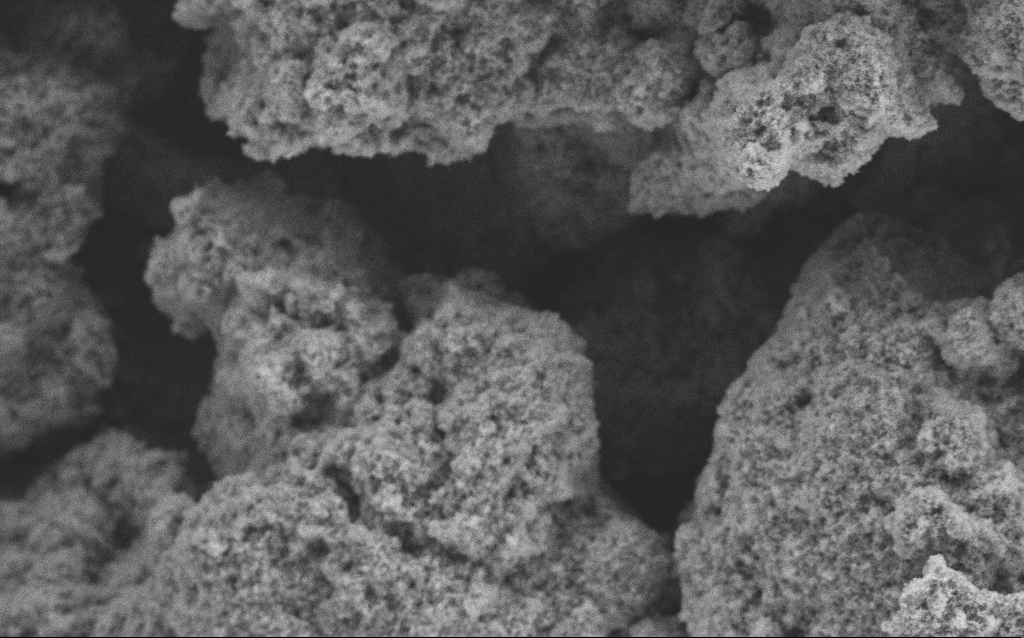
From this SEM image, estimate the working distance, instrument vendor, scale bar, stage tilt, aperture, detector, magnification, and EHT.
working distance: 4.1 mm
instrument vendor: Zeiss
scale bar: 10000 nm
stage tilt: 0°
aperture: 30 µm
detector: SE2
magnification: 6.41 K X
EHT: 5 kV